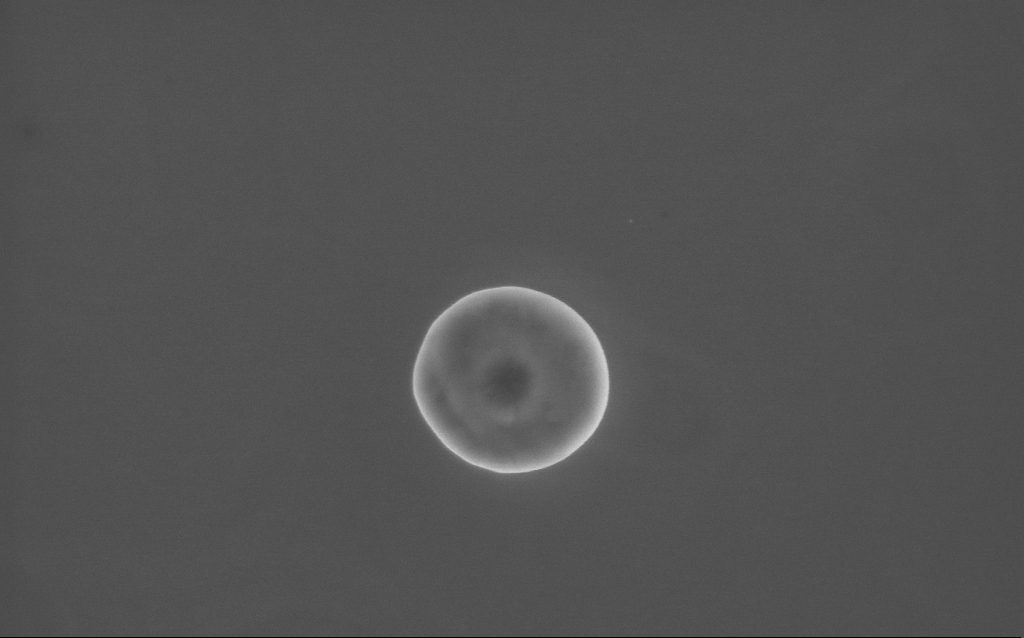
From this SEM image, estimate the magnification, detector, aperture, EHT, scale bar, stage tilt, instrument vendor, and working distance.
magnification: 57.36 K X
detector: InLens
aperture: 30 µm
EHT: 10 kV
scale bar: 1000 nm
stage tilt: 0°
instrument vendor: Zeiss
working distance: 2 mm